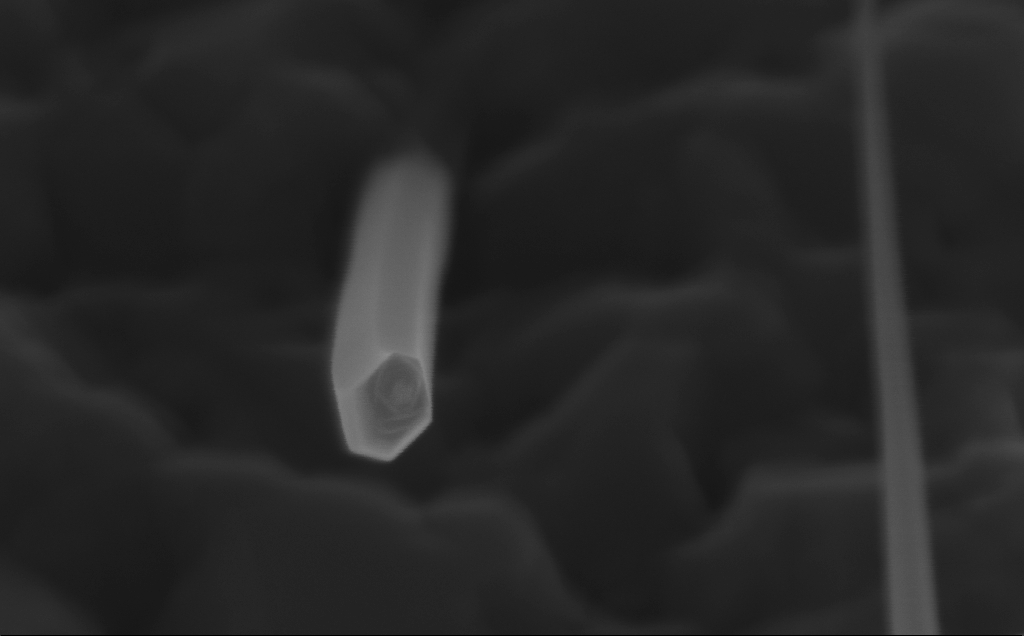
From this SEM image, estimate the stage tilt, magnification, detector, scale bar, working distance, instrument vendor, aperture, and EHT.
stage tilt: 45°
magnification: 323.33 K X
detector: InLens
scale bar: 200 nm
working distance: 5 mm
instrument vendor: Zeiss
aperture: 30 µm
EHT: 10 kV